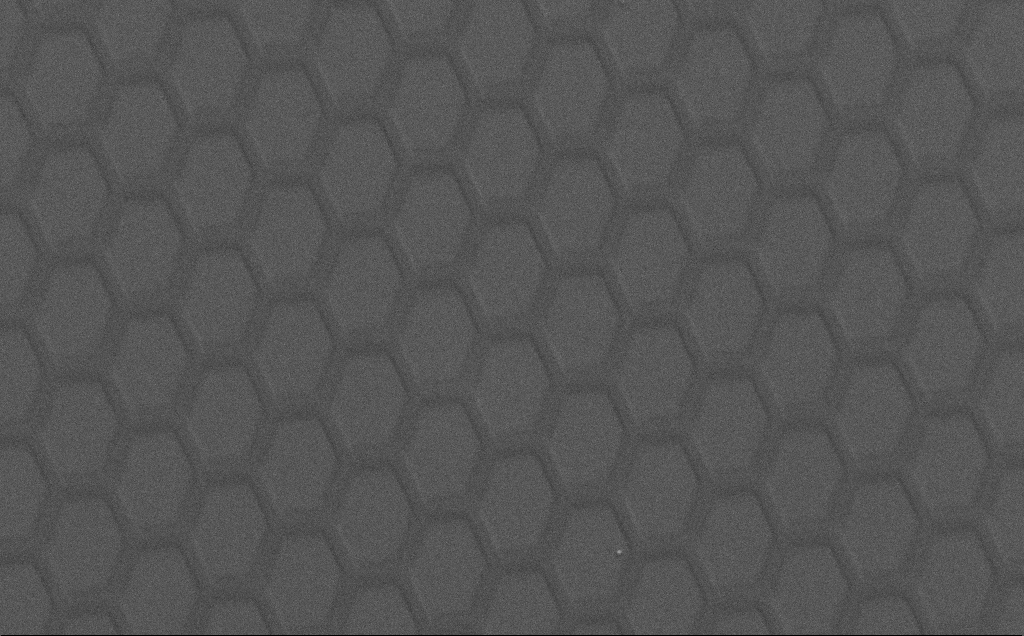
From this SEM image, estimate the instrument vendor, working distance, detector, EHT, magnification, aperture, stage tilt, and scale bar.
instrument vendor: Zeiss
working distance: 5 mm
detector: SE2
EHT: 1.5 kV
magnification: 0.507 K X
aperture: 30 µm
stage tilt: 0°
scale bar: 100000 nm